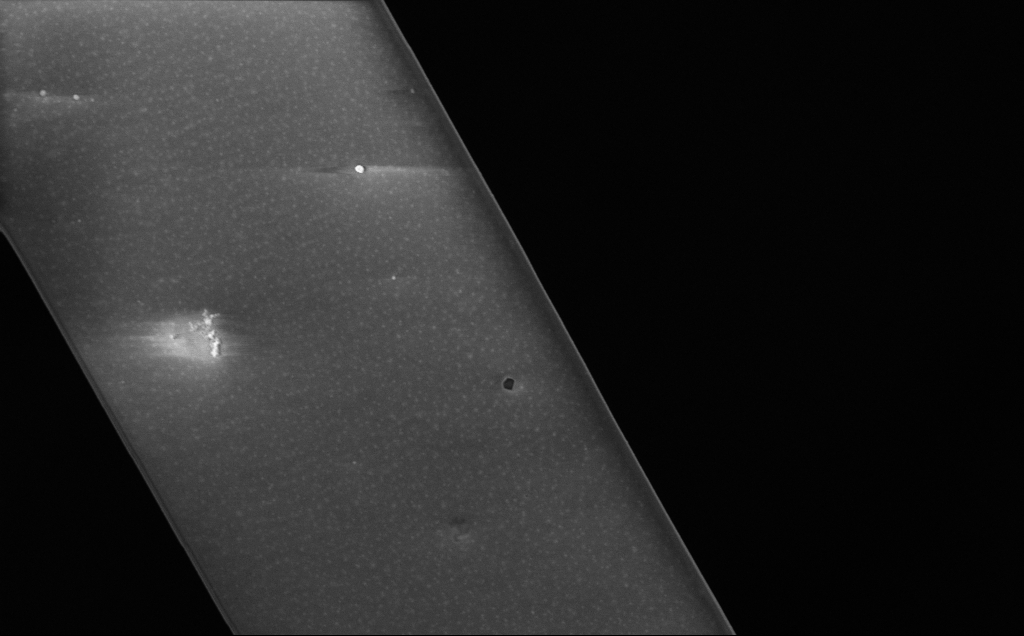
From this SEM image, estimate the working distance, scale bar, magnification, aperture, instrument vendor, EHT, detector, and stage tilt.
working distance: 10 mm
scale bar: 10000 nm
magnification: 4.26 K X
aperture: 30 µm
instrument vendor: Zeiss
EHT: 5 kV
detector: InLens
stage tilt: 0°